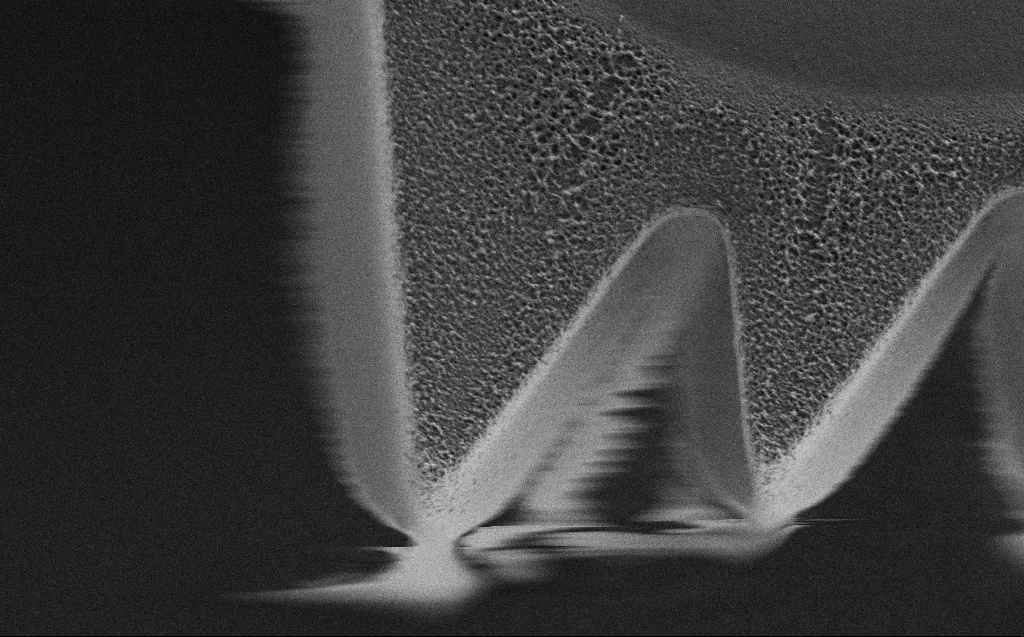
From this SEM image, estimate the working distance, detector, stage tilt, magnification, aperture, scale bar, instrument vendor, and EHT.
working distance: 4 mm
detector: SE2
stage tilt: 0°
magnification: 10.92 K X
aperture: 30 µm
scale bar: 2000 nm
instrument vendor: Zeiss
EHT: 1 kV